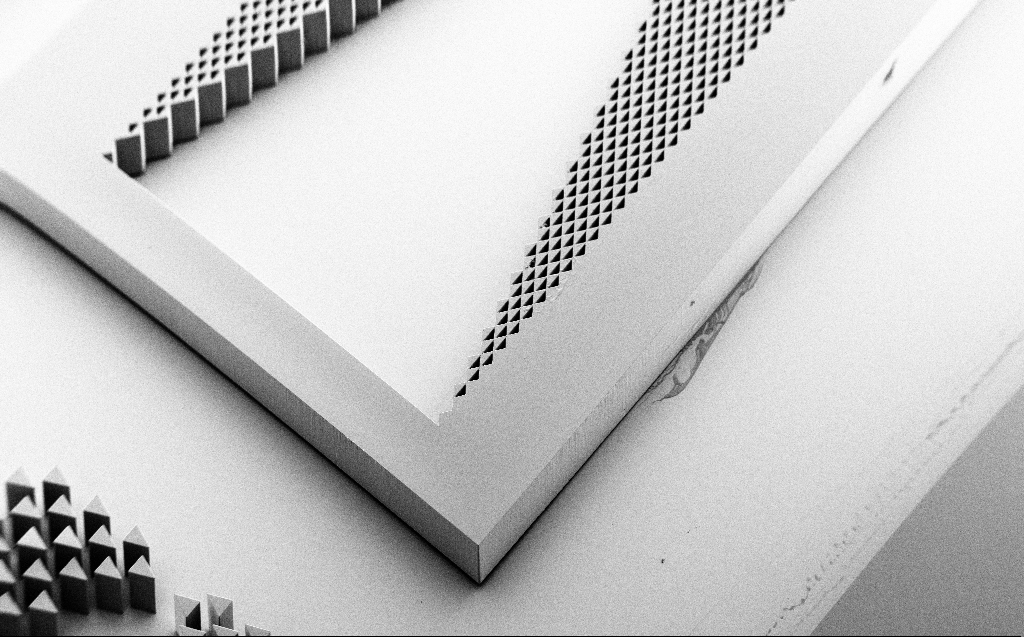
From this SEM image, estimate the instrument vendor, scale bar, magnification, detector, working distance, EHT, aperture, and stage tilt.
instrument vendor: Zeiss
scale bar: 200000 nm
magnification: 0.081 K X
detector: SE2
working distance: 10 mm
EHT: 5 kV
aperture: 30 µm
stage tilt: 45°